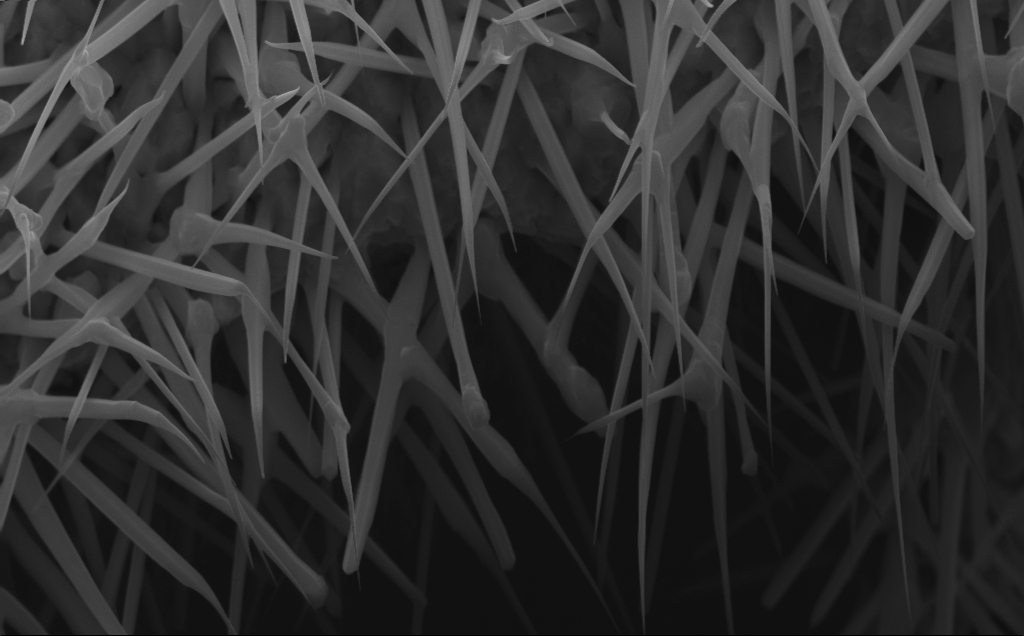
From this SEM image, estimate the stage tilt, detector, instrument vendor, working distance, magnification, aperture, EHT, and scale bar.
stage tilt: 0°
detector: InLens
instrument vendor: Zeiss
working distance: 7 mm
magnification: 40 K X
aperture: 30 µm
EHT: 10 kV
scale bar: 1000 nm